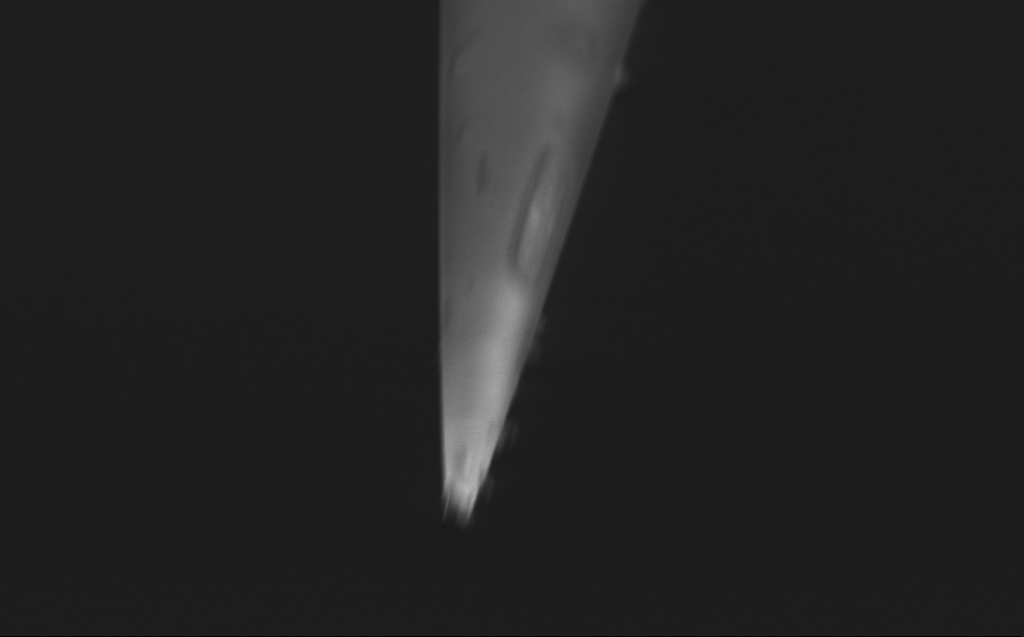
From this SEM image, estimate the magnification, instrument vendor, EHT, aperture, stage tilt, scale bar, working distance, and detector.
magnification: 53.49 K X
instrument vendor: Zeiss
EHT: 1 kV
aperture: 30 µm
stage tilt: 45°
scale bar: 1000 nm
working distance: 3 mm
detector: InLens